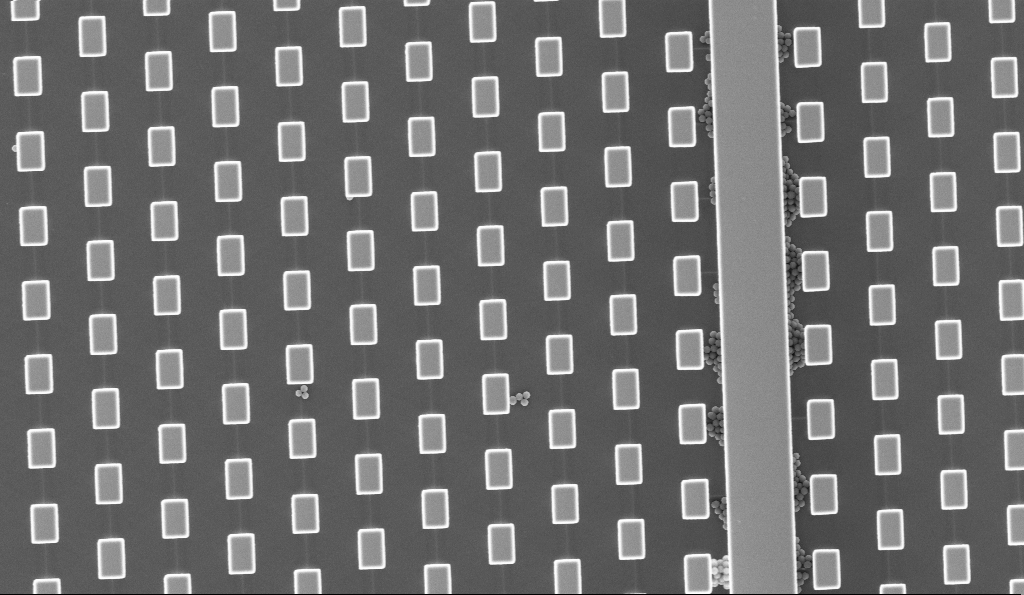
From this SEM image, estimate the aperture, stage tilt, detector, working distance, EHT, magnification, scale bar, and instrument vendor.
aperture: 30 µm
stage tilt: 0°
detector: InLens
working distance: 3.1 mm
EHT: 5 kV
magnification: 4.59 K X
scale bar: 10000 nm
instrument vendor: Zeiss